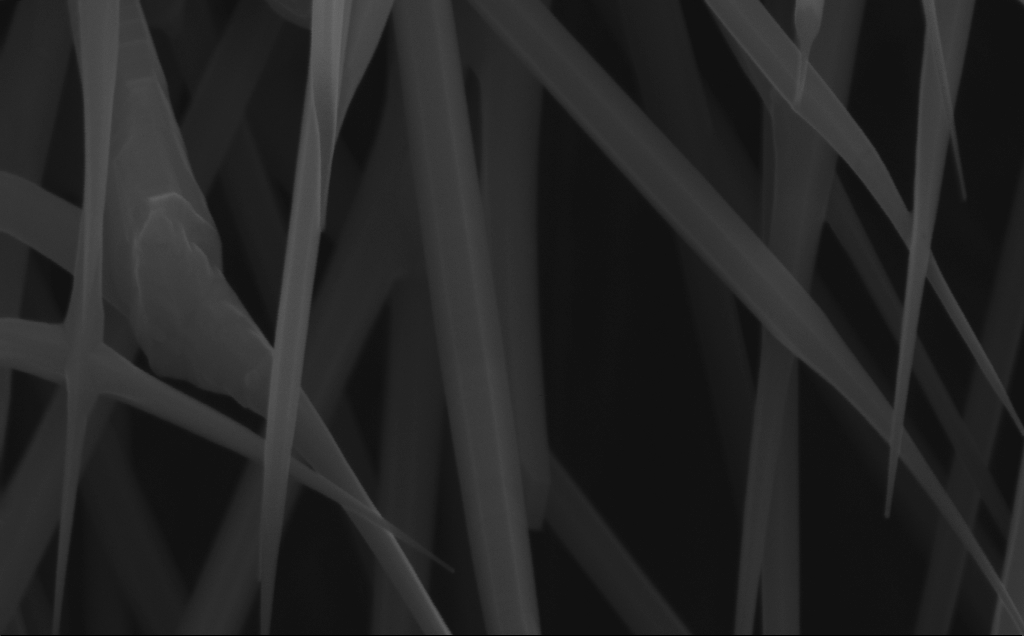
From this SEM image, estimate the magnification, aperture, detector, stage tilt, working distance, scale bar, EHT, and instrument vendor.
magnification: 127.73 K X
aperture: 30 µm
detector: InLens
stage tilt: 0°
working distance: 7 mm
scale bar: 200 nm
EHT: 10 kV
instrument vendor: Zeiss